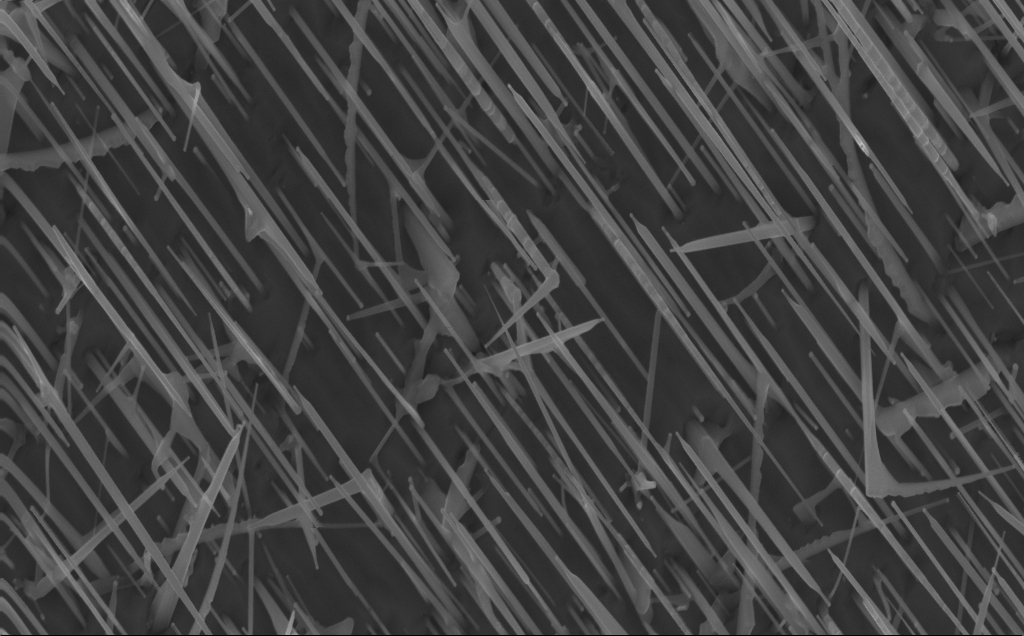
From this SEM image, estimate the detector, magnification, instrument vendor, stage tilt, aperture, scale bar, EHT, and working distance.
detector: InLens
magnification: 40 K X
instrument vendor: Zeiss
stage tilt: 0°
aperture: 30 µm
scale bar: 1000 nm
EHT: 10 kV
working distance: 4 mm